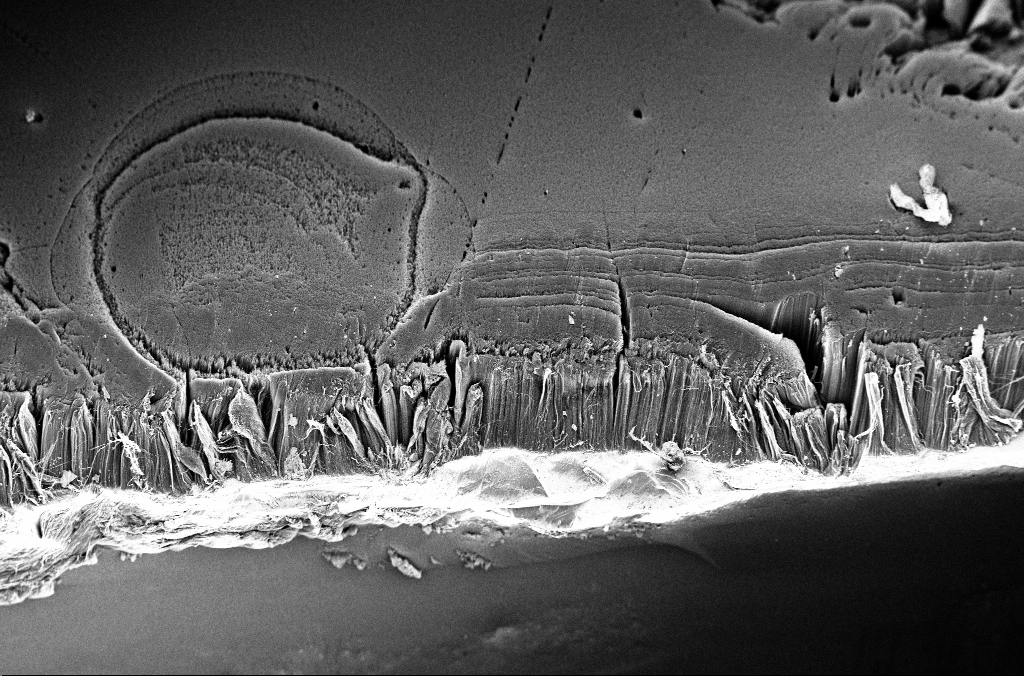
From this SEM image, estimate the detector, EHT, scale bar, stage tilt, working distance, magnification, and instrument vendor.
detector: InLens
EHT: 3 kV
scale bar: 100000 nm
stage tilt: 45°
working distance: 3.3 mm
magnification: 0.3 K X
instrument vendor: Zeiss